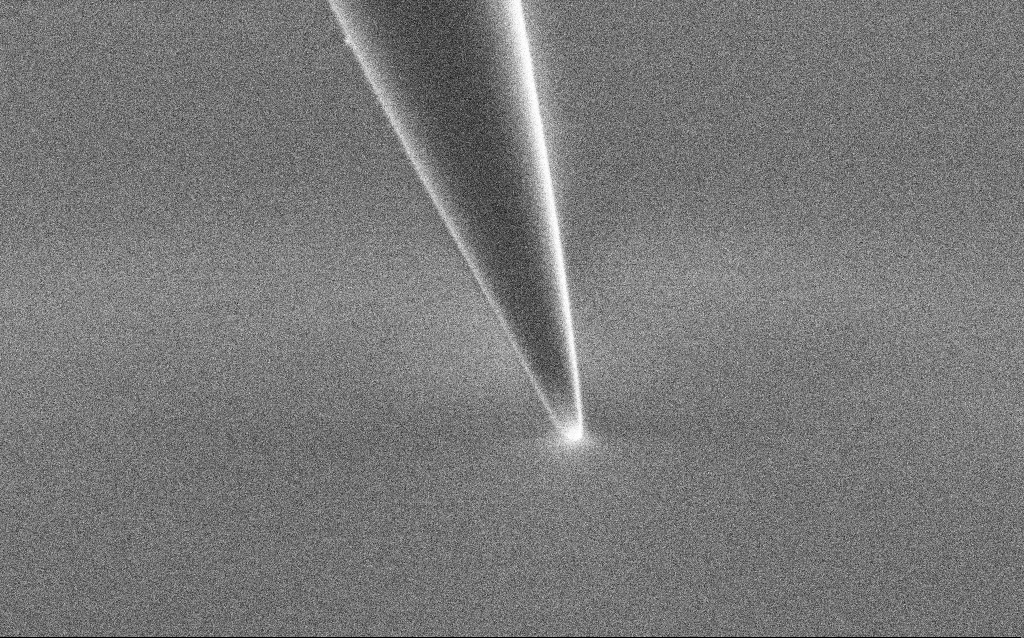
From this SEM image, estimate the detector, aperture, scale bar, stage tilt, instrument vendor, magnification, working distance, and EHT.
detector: SE2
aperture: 30 µm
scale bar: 1000 nm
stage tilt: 45°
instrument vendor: Zeiss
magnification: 50 K X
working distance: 7 mm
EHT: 1 kV